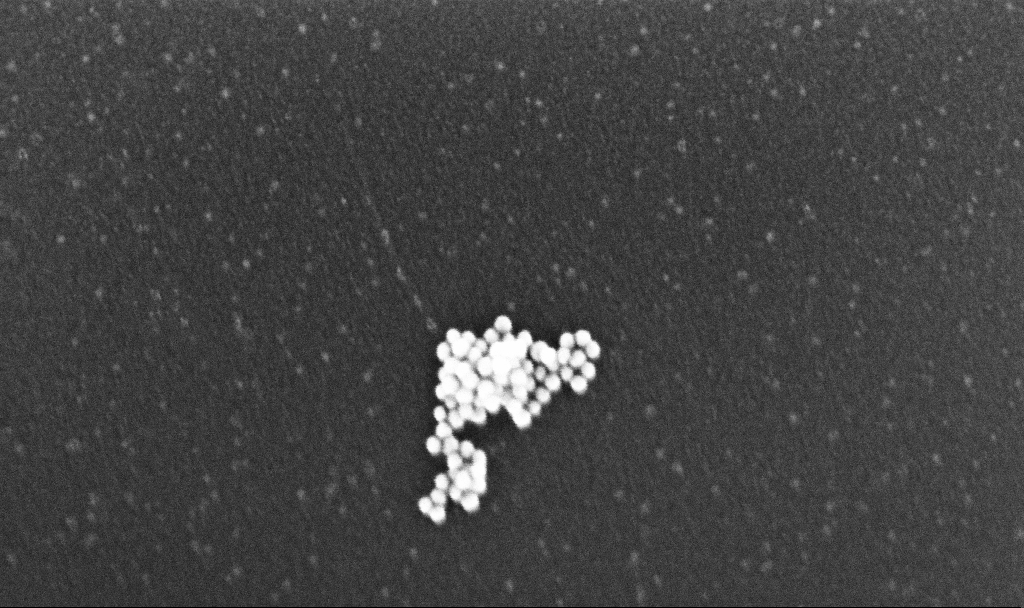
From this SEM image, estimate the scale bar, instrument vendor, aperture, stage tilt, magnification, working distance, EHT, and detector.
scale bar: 200 nm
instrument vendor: Zeiss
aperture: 30 µm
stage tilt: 0°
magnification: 263.55 K X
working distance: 3.1 mm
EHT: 10 kV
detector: InLens